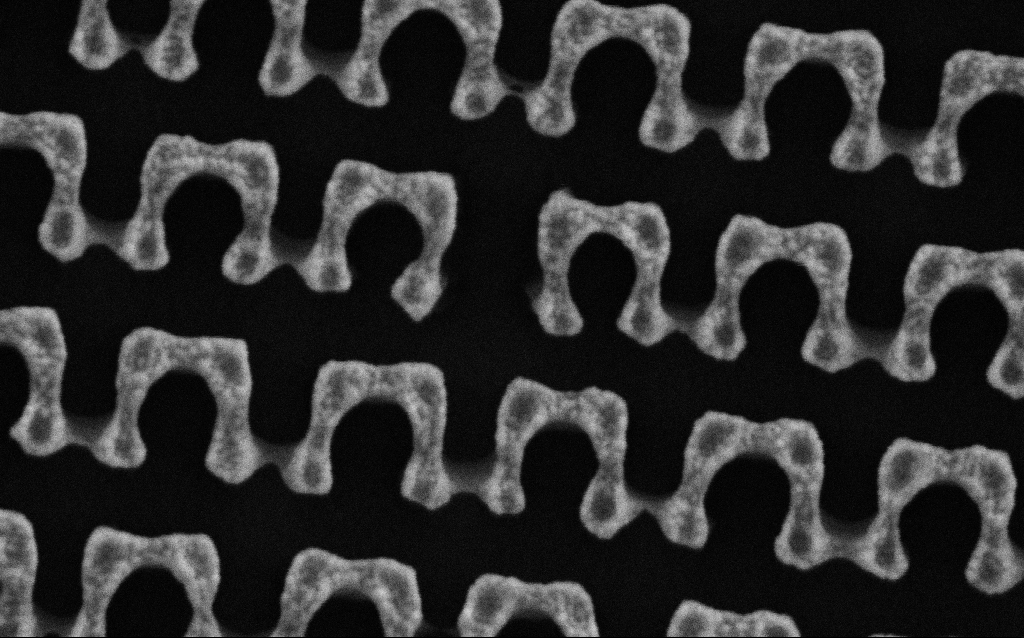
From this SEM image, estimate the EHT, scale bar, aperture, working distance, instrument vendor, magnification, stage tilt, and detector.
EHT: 3 kV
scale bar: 200 nm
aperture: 30 µm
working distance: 4.6 mm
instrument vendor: Zeiss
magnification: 155 K X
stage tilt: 0°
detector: SE2